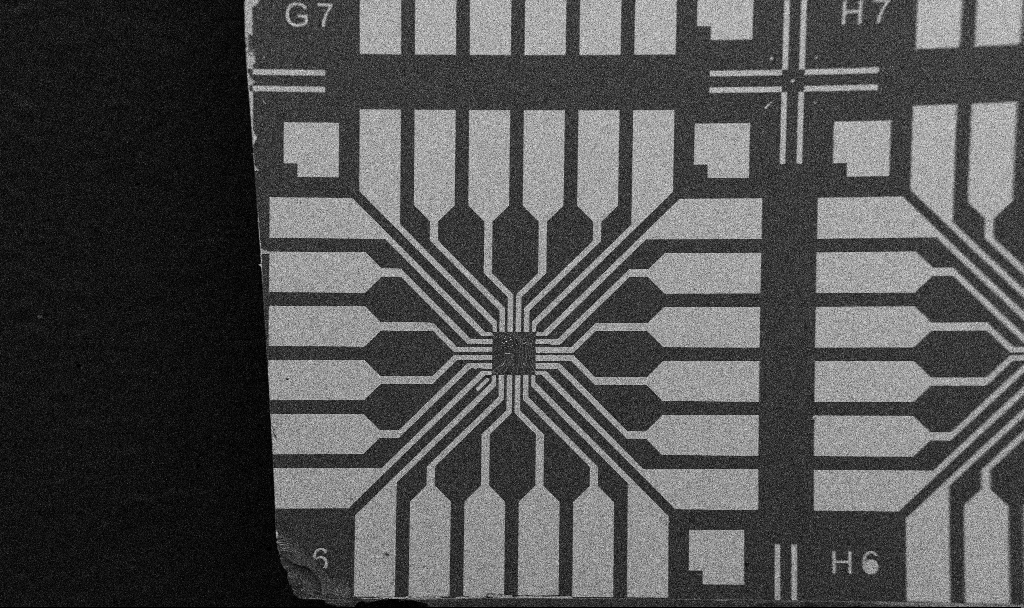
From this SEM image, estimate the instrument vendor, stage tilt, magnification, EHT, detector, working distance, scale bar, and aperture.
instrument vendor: Zeiss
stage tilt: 0°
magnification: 0.1 K X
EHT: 5 kV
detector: SE2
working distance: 10.7 mm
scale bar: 200000 nm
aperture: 30 µm